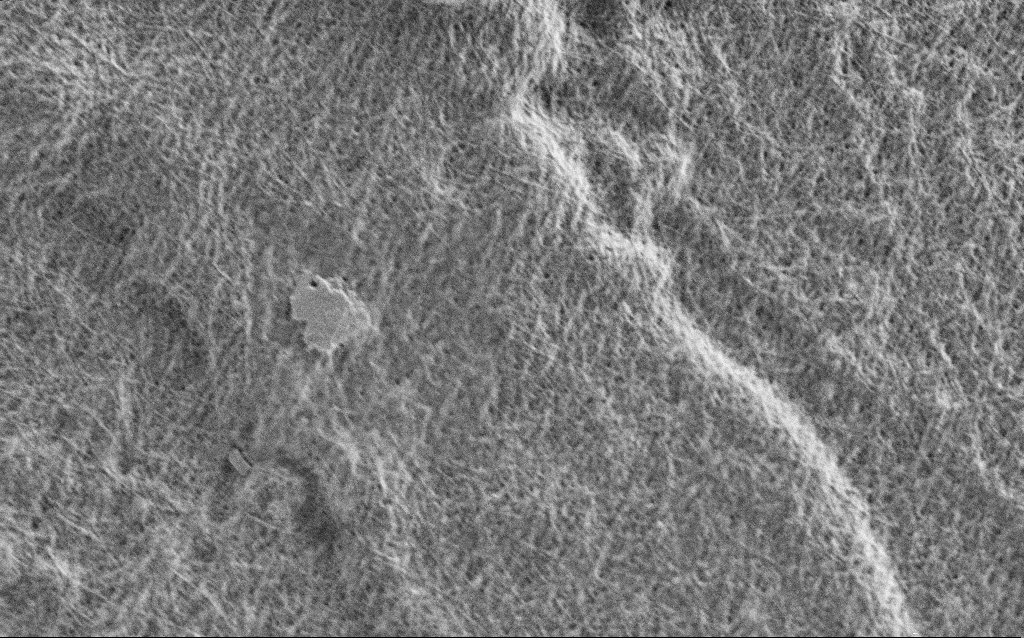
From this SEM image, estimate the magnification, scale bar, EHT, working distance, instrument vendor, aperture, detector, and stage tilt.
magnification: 20 K X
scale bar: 1000 nm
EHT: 1 kV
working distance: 4 mm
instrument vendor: Zeiss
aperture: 30 µm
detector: SE2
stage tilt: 0°